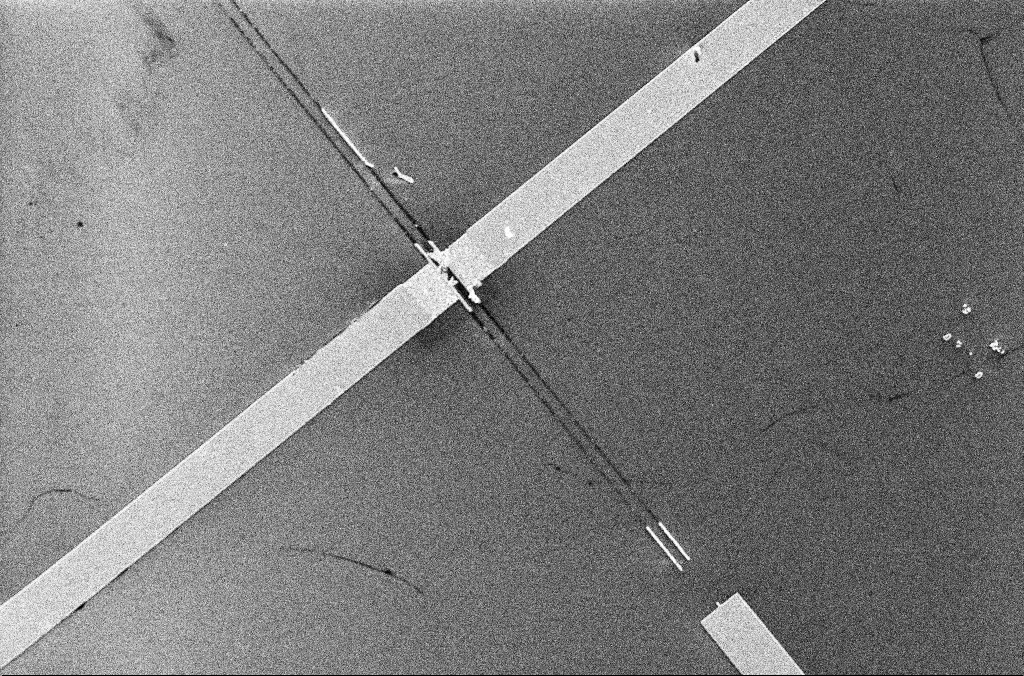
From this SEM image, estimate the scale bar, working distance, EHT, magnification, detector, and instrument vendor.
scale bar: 10000 nm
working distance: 3.4 mm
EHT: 5 kV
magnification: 3.49 K X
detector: InLens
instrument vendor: Zeiss